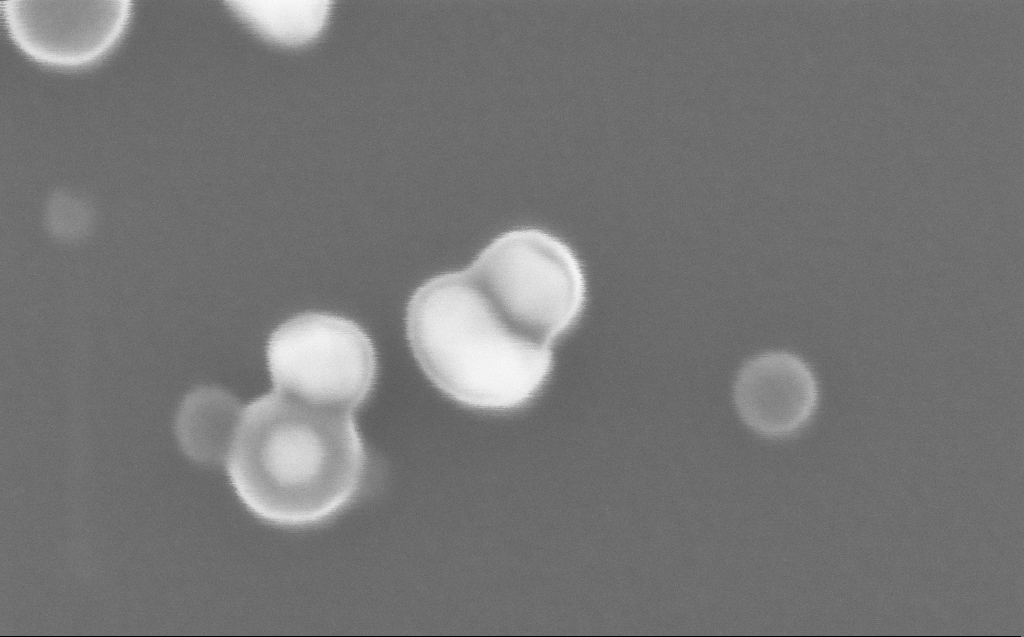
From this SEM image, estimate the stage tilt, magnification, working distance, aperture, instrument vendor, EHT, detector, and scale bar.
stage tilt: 0°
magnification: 800 K X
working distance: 3 mm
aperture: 30 µm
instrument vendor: Zeiss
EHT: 10 kV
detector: InLens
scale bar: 20 nm